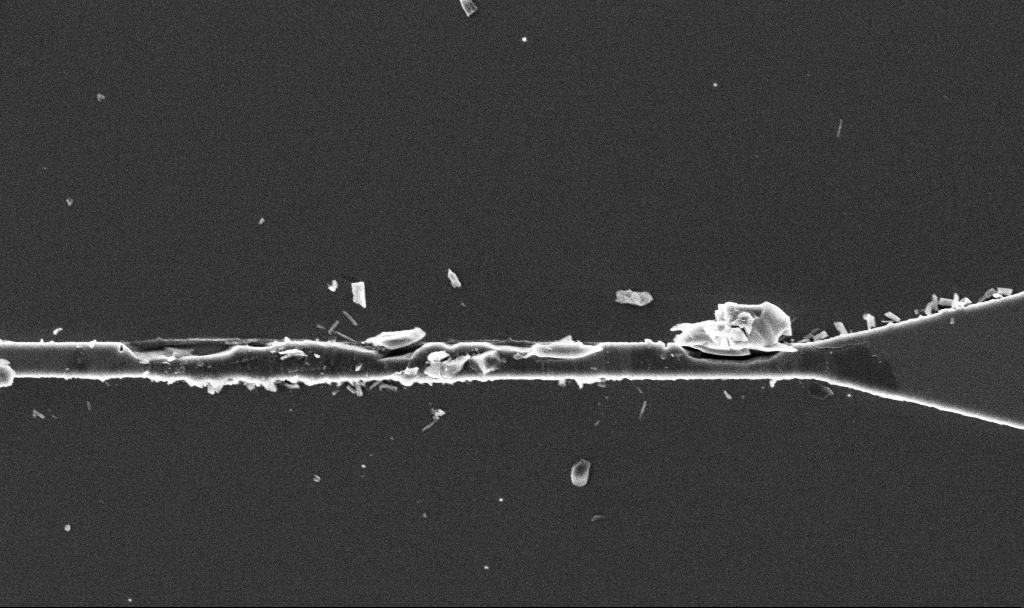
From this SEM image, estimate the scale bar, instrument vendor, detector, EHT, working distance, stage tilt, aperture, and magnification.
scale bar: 2000 nm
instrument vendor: Zeiss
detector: InLens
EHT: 3 kV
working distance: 2.9 mm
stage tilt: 0°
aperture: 30 µm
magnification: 24.65 K X